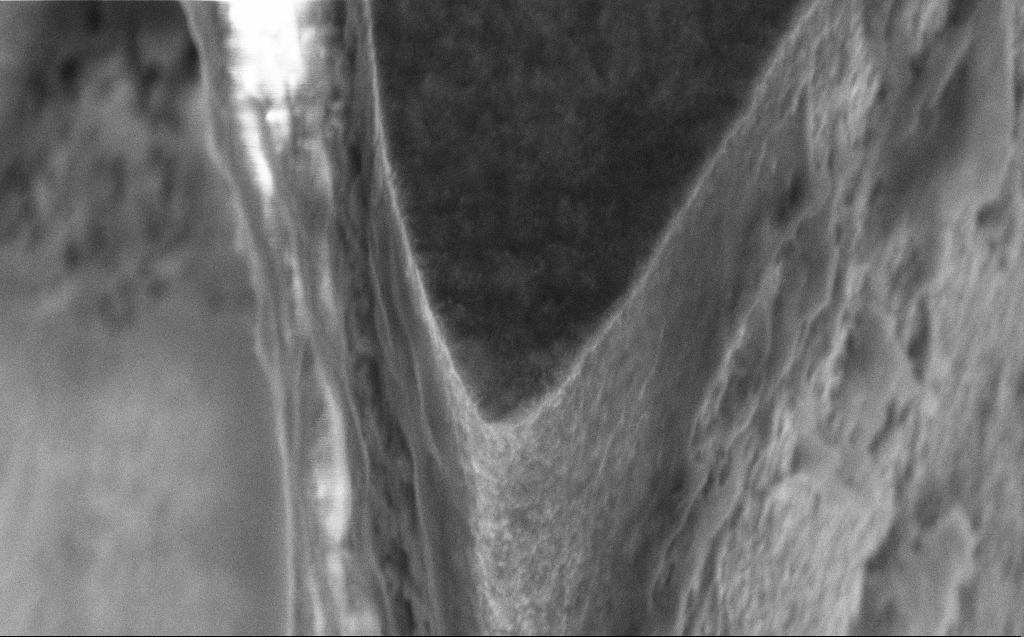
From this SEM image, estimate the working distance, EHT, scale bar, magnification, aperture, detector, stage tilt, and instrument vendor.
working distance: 7 mm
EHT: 5 kV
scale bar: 1000 nm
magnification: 48.41 K X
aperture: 30 µm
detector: InLens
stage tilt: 45°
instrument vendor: Zeiss